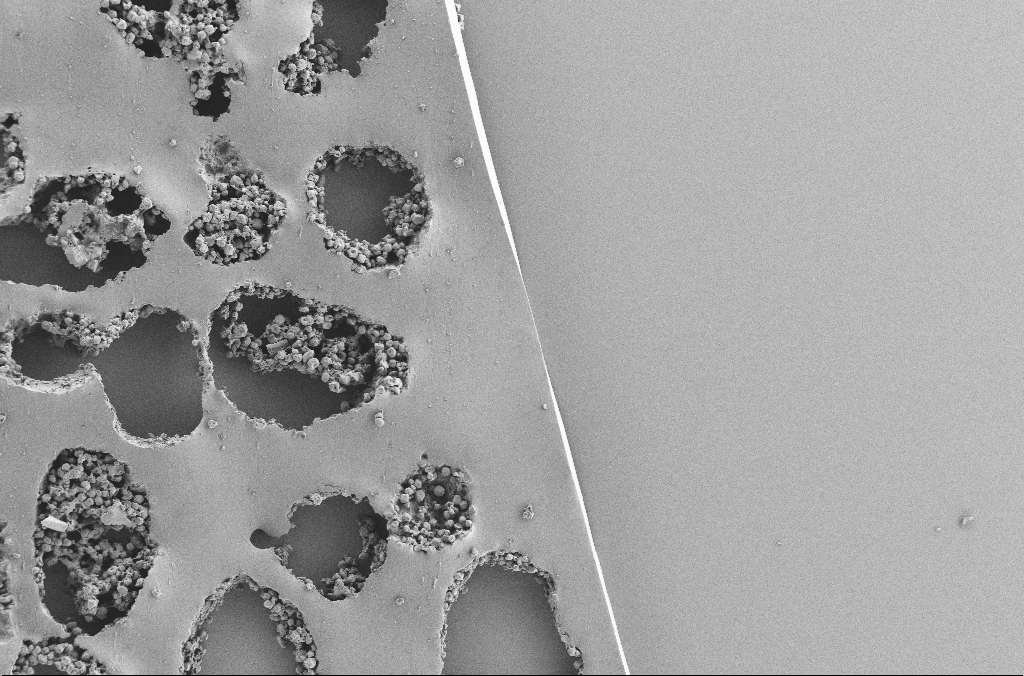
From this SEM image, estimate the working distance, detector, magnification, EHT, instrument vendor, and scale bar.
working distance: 3.7 mm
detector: SE2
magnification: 0.25 K X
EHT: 2 kV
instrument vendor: Zeiss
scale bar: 100000 nm